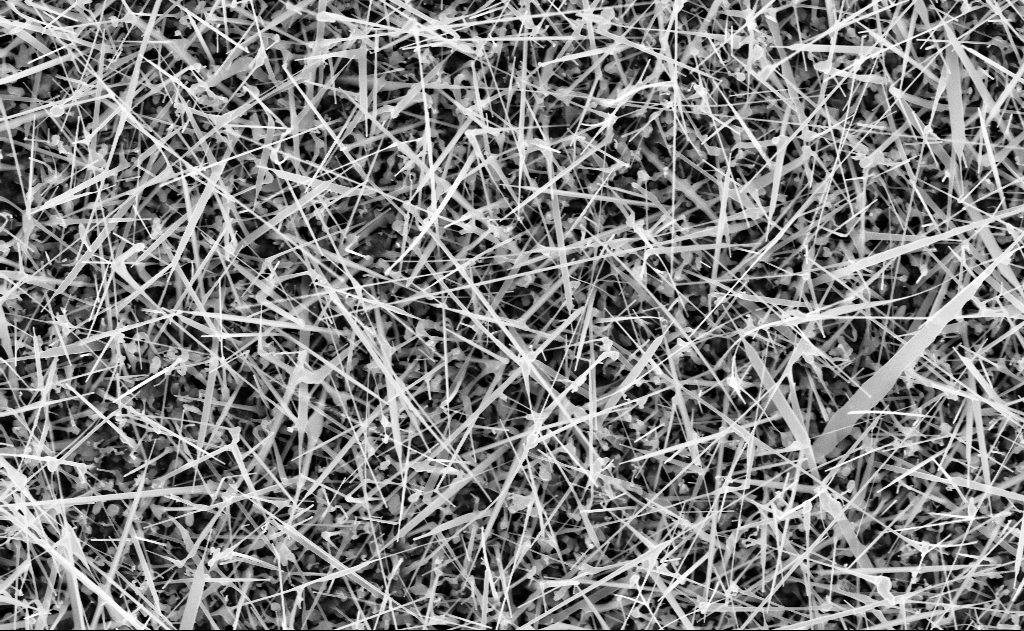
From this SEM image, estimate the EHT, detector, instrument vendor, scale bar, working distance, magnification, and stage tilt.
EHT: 10 kV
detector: InLens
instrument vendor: Zeiss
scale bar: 1000 nm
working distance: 11 mm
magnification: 20 K X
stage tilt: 0°